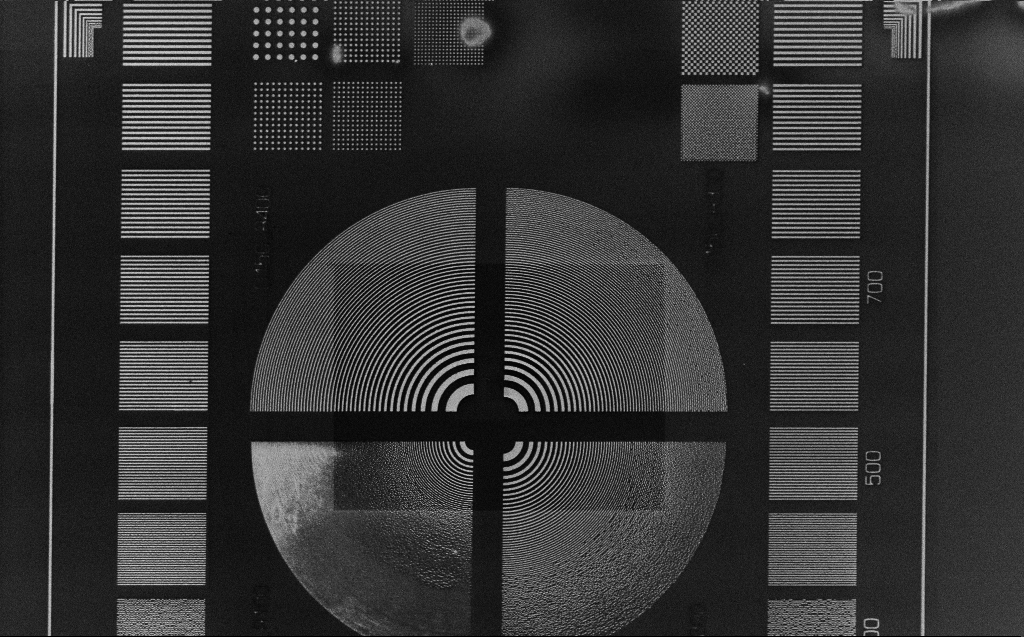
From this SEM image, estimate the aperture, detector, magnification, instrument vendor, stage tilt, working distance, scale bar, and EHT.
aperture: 30 µm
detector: InLens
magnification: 1.1 K X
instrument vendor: Zeiss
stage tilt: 0°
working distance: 4 mm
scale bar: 20000 nm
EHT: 3 kV